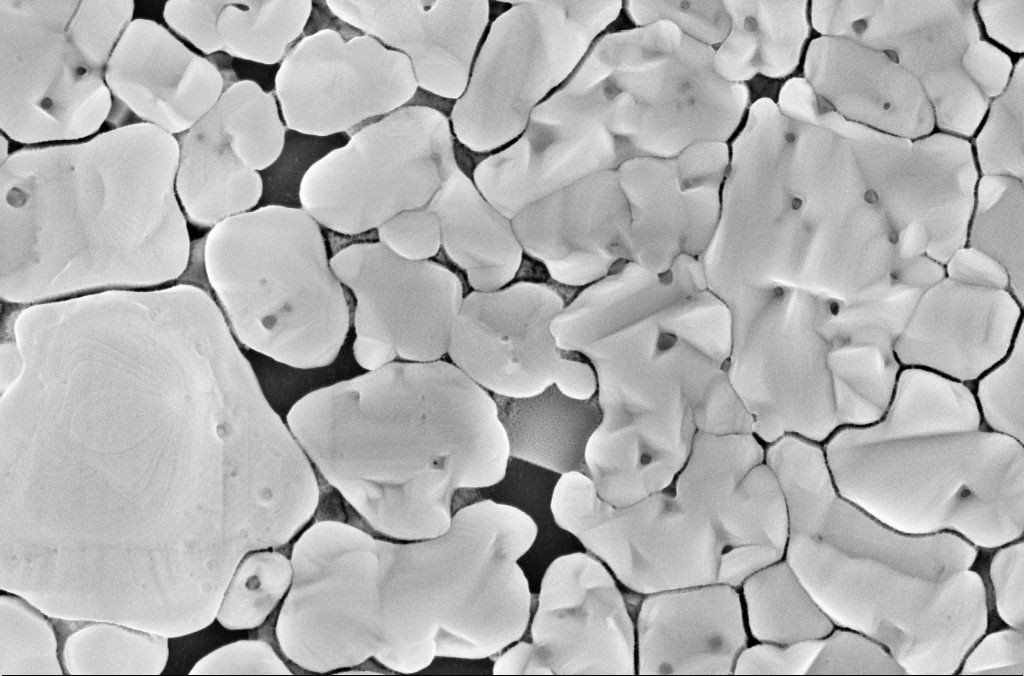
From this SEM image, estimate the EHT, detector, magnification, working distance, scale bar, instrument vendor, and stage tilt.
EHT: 5 kV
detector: InLens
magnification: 70 K X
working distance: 3 mm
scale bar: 200 nm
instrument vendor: Zeiss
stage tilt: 0°